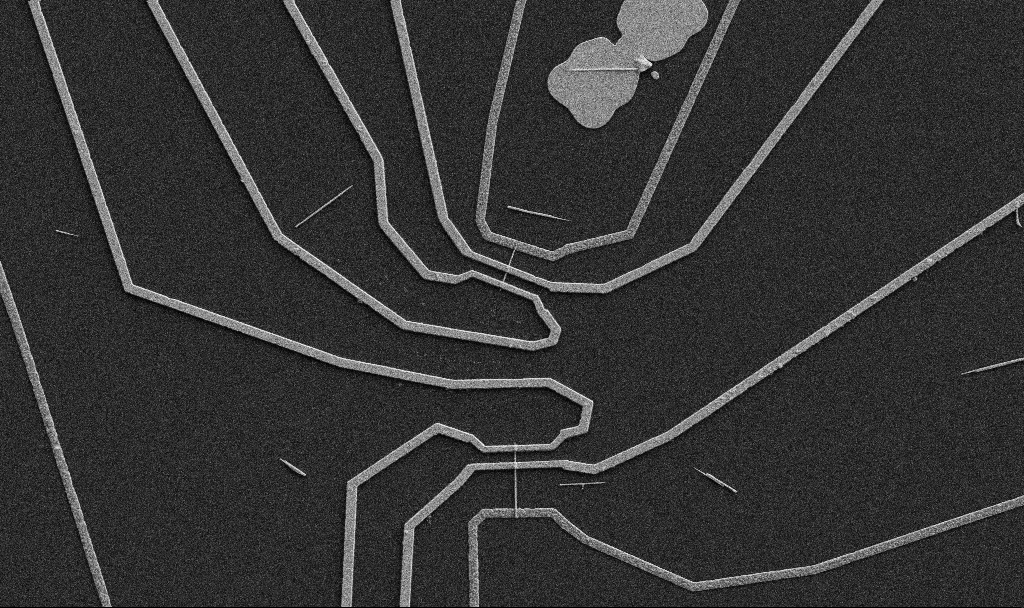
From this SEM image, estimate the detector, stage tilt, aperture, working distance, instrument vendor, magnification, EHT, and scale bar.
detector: SE2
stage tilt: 0°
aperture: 30 µm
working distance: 10.7 mm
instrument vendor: Zeiss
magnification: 5 K X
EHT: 5 kV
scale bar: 10000 nm